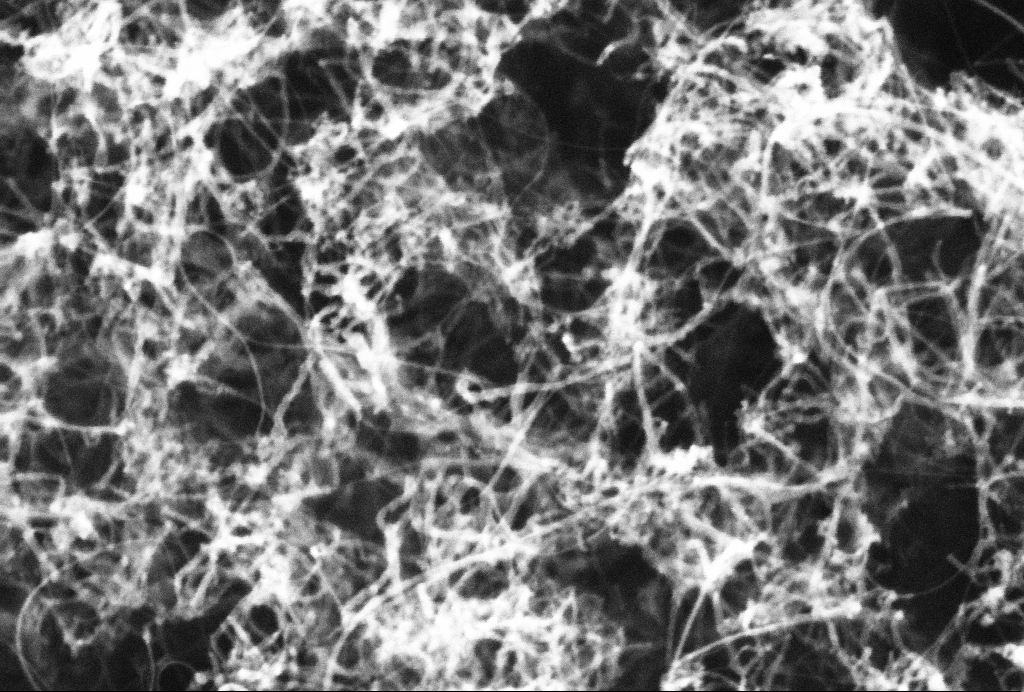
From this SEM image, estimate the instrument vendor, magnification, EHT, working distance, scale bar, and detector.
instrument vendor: Zeiss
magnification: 336.4 K X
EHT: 10 kV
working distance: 2.9 mm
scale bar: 100 nm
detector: InLens